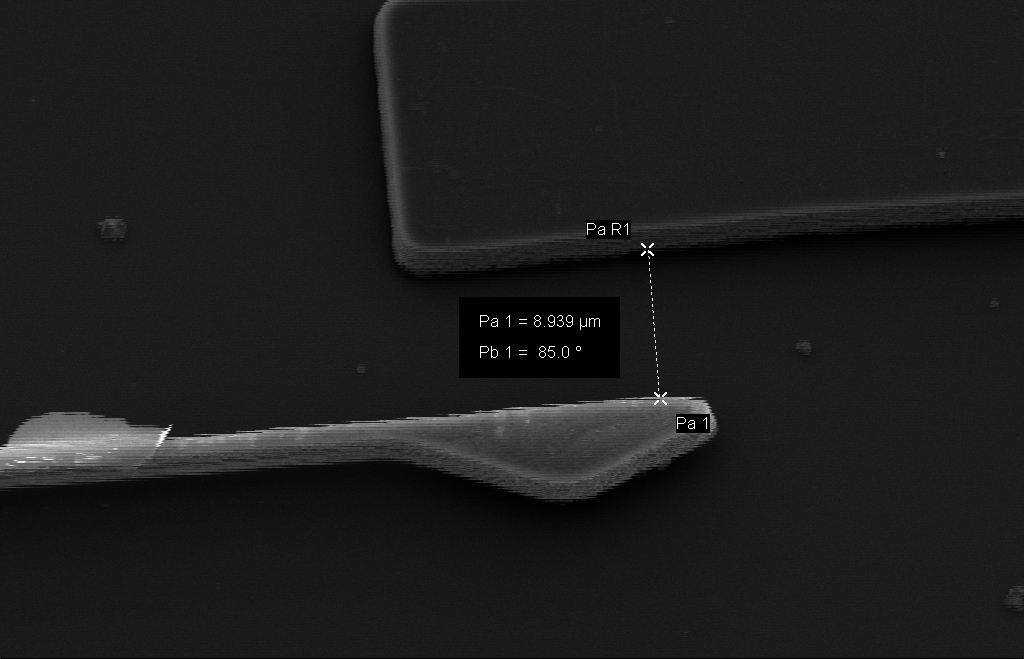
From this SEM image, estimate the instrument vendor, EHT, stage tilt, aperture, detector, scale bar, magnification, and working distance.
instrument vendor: Zeiss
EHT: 10 kV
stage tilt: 23.3°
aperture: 30 µm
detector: SE2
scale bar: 2000 nm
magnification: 6.14 K X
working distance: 20 mm